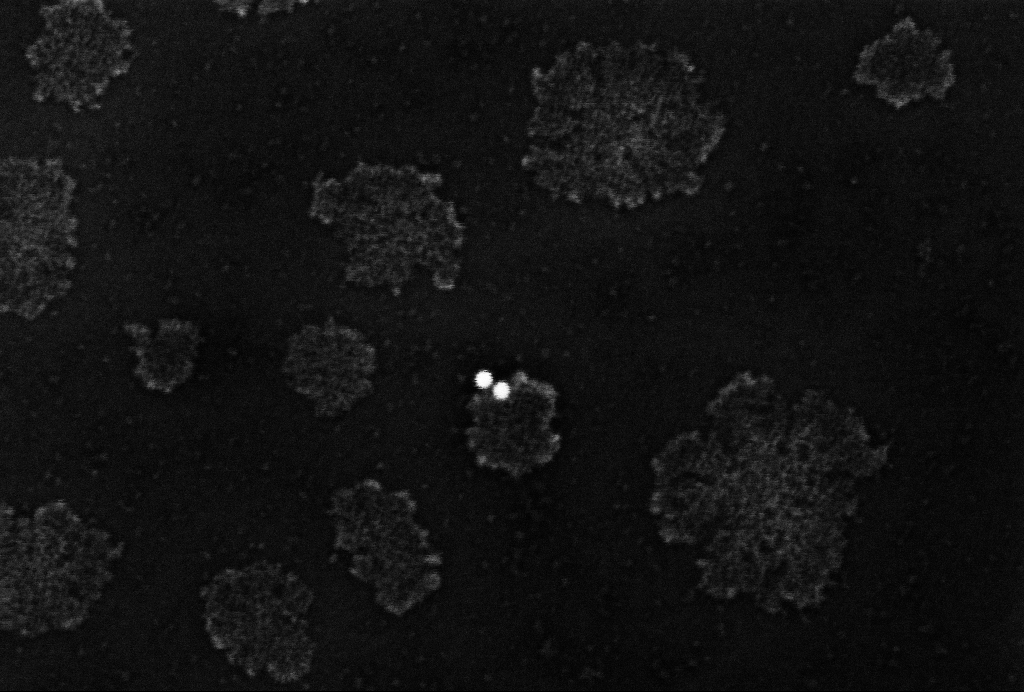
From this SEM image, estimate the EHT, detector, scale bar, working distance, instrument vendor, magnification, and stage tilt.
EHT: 2 kV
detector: InLens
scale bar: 200 nm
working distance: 3.3 mm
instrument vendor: Zeiss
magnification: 301.52 K X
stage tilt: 0°